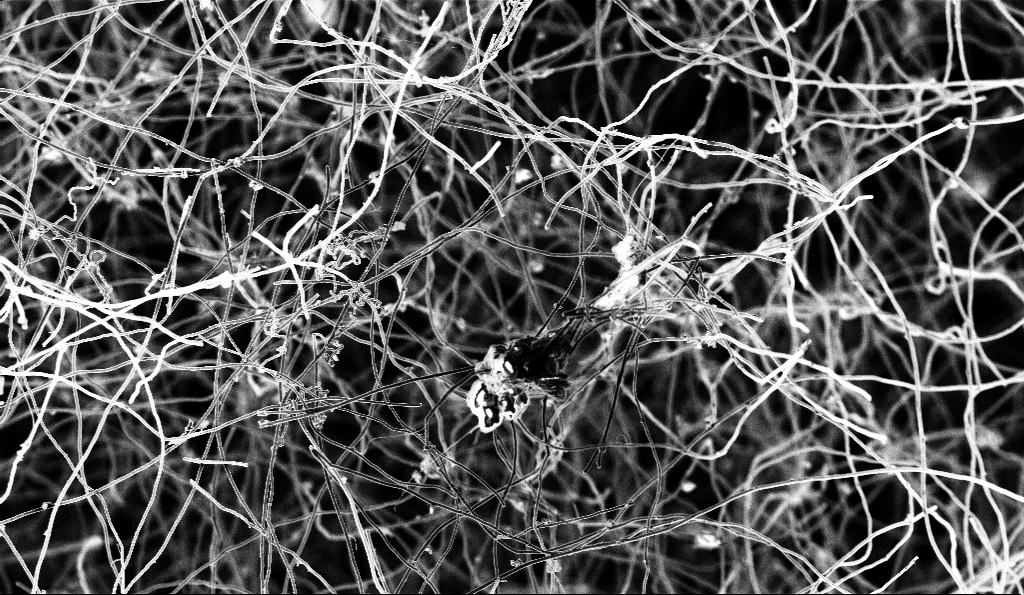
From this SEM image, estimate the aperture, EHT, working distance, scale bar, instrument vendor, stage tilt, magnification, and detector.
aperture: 30 µm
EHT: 3 kV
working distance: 5.5 mm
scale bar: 10000 nm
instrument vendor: Zeiss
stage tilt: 0°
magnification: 2 K X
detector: InLens